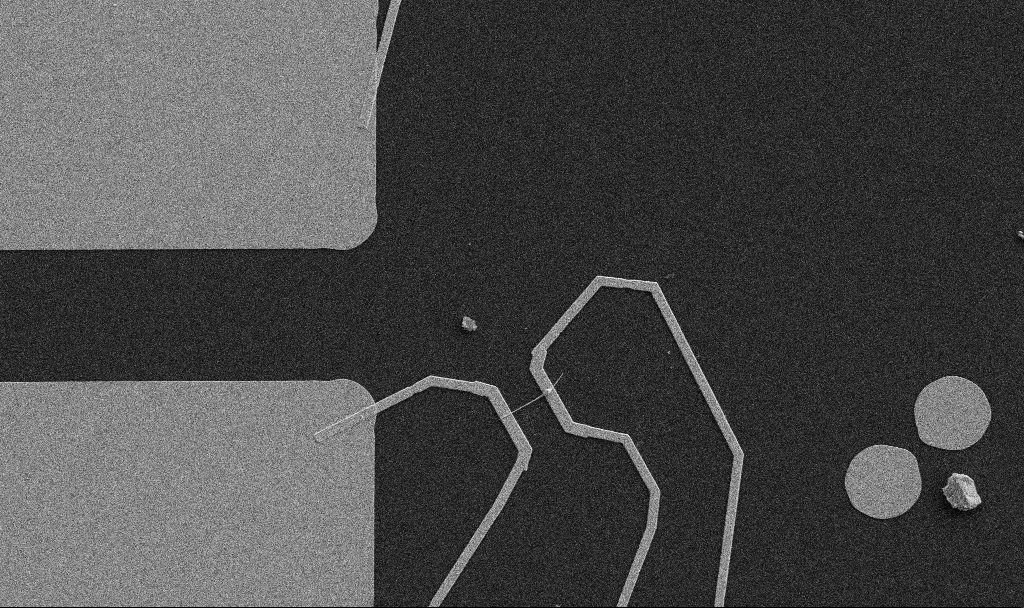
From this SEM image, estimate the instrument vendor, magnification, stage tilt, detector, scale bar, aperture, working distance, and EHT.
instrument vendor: Zeiss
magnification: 5 K X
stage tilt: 0°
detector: SE2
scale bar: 10000 nm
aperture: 30 µm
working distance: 10.7 mm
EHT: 5 kV